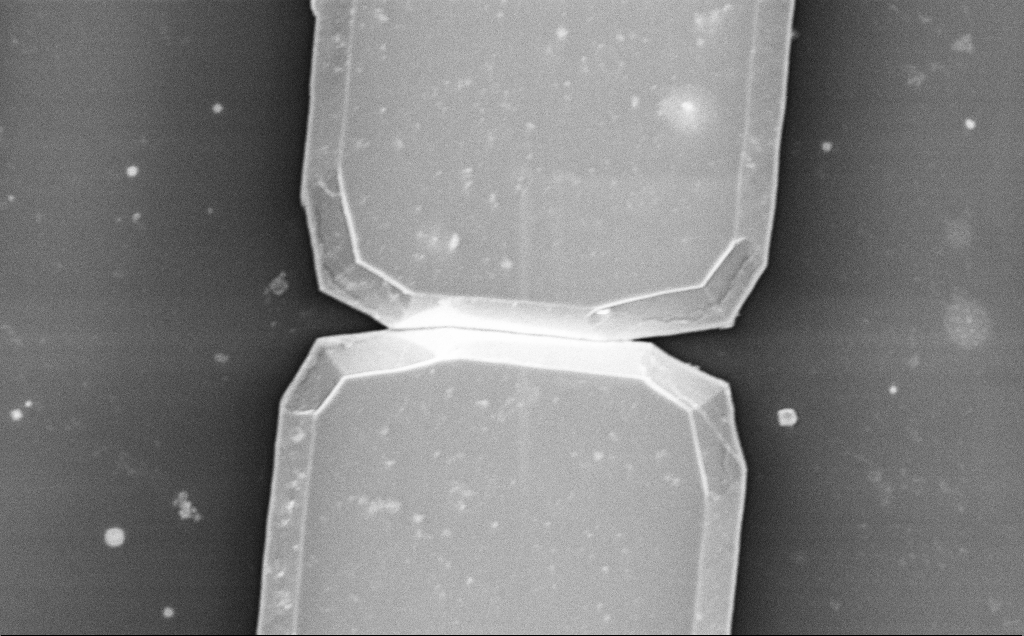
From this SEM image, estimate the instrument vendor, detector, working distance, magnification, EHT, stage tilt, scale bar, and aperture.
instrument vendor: Zeiss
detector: InLens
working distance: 14 mm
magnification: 7.68 K X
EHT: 5 kV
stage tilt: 0°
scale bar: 2000 nm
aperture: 30 µm